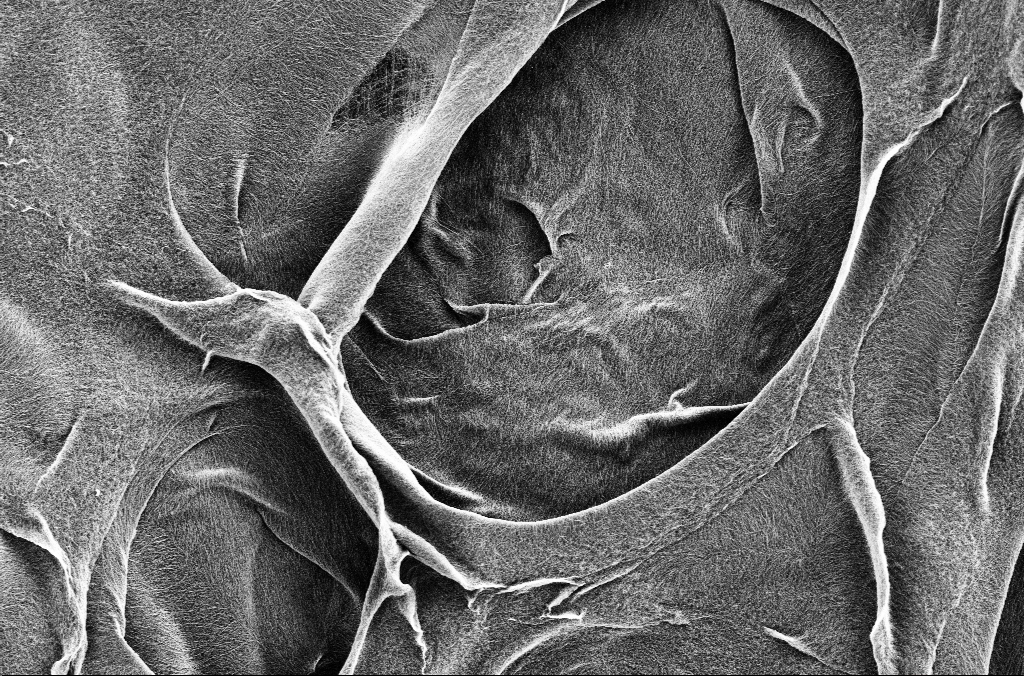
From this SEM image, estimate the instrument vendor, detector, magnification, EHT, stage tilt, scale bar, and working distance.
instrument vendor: Zeiss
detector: SE2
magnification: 5 K X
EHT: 1.8 kV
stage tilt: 0°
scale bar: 10000 nm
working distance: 5.7 mm